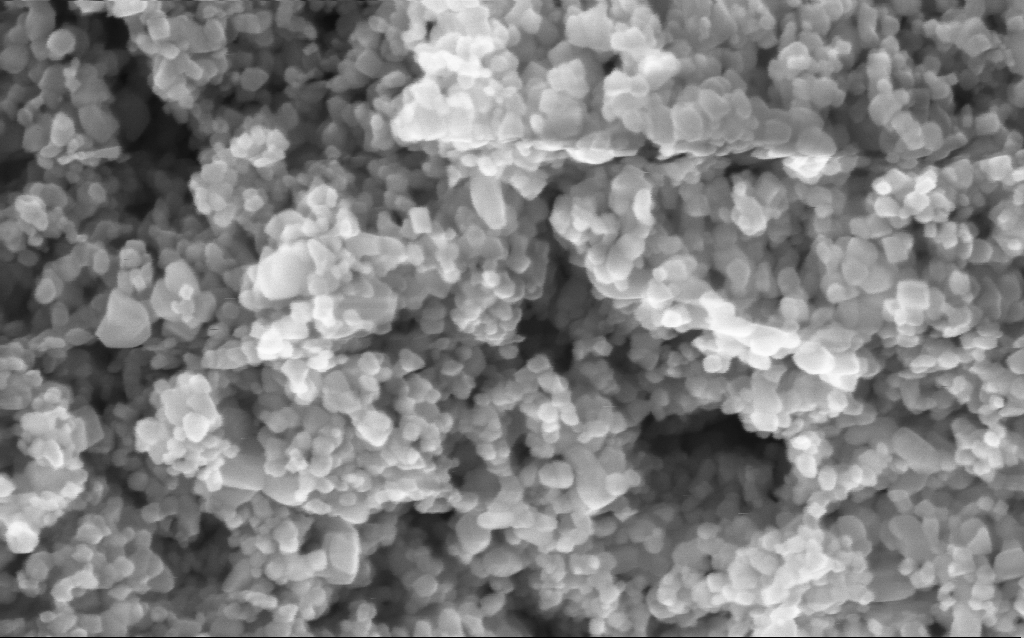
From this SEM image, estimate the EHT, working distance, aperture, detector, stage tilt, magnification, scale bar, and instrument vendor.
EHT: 5 kV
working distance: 4.4 mm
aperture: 30 µm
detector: InLens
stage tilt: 0°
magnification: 294.5 K X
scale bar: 200 nm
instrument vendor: Zeiss